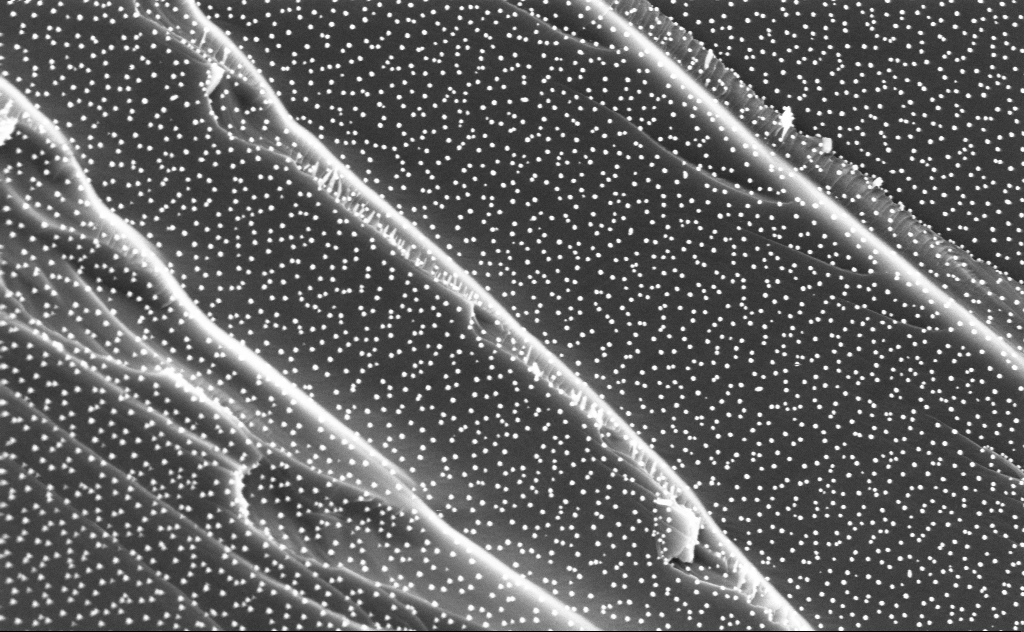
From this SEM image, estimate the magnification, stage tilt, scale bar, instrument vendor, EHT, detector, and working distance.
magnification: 80 K X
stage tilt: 0°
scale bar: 200 nm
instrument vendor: Zeiss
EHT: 3 kV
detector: InLens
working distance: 5 mm